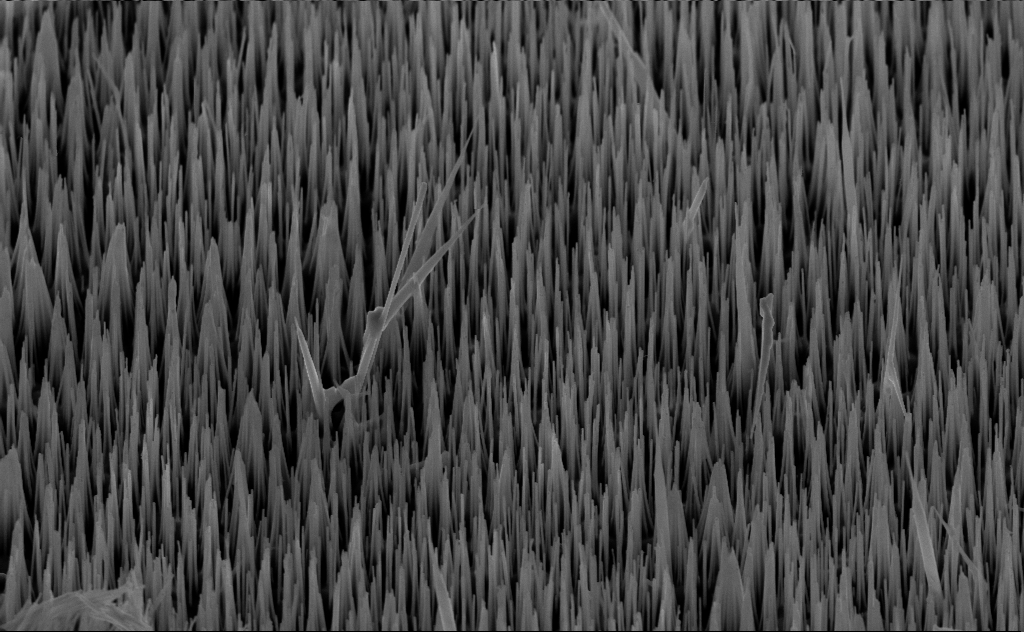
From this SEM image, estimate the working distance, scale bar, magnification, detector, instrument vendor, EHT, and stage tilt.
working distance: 6 mm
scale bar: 2000 nm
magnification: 20 K X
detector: InLens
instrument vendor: Zeiss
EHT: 10 kV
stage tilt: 45°